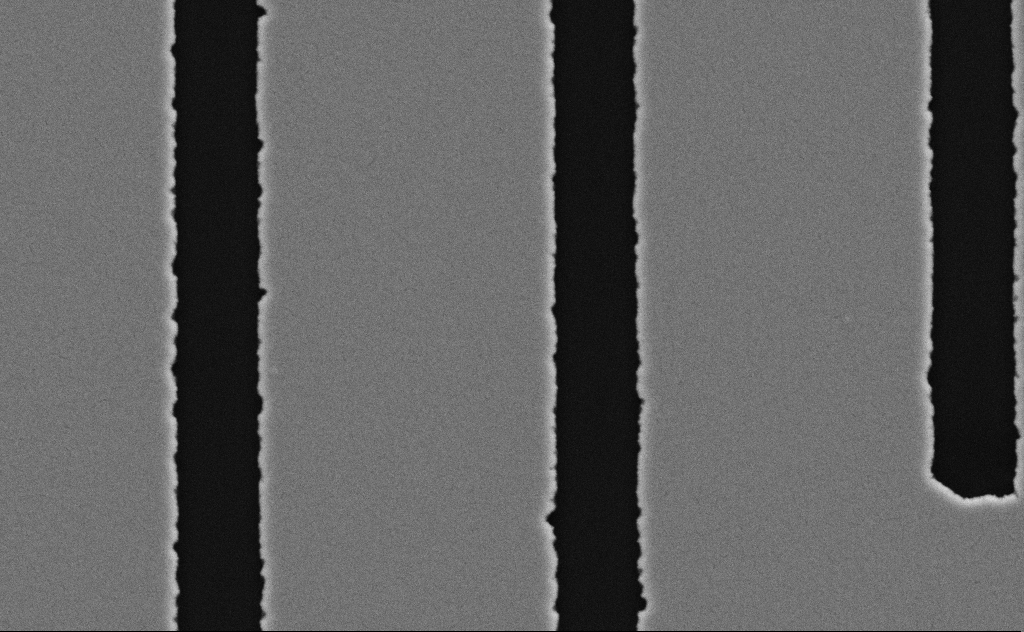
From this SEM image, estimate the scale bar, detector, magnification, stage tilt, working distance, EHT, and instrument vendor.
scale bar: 1000 nm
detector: SE2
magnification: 34.66 K X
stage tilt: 0°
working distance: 8 mm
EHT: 5 kV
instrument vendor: Zeiss